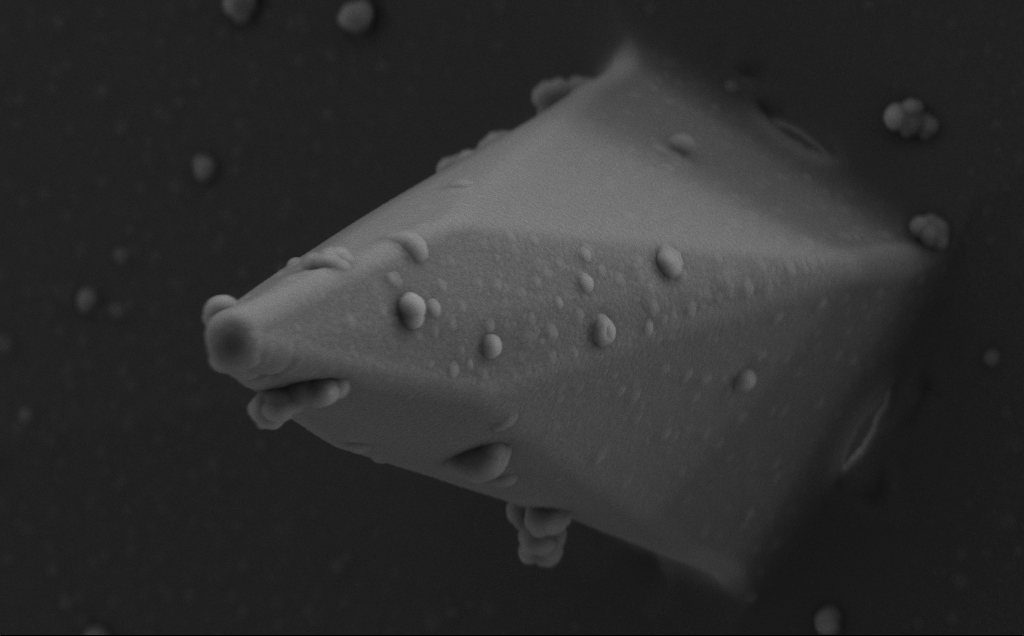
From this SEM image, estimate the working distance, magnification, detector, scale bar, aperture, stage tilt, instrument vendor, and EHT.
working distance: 8 mm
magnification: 24.76 K X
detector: SE2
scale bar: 2000 nm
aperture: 30 µm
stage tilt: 0°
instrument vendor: Zeiss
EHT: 5 kV